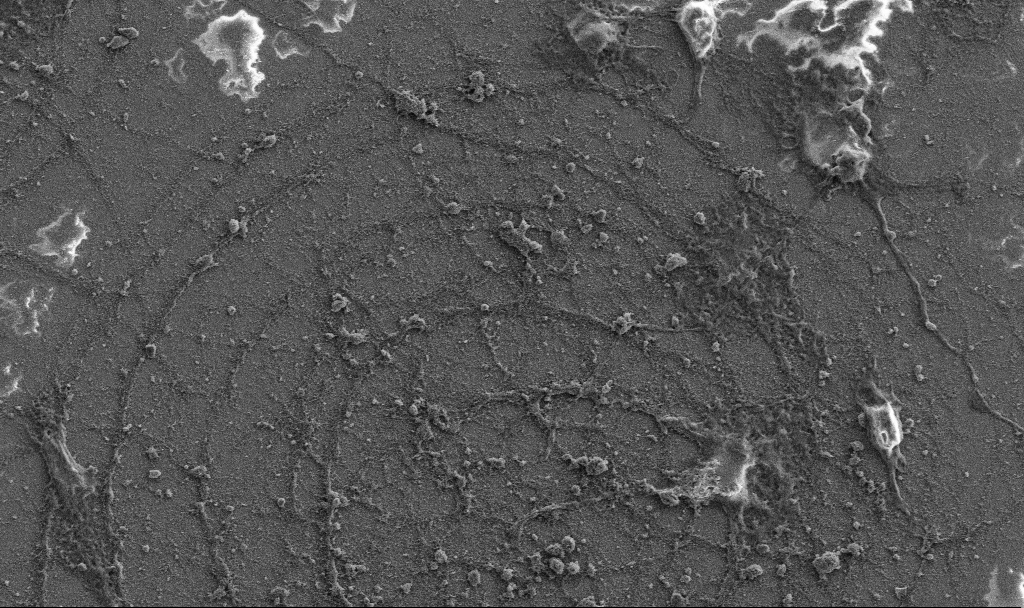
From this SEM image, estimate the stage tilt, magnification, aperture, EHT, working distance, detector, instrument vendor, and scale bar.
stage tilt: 0°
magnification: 2 K X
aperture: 30 µm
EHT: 5 kV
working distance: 6.8 mm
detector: SE2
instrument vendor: Zeiss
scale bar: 10000 nm